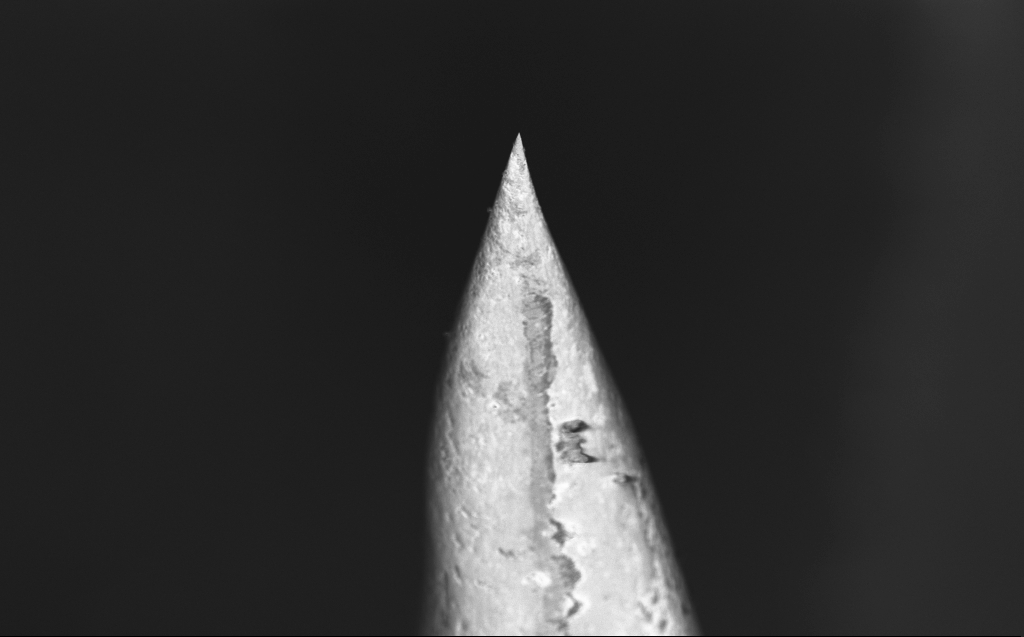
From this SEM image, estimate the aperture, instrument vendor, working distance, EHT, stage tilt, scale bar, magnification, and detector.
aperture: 30 µm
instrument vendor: Zeiss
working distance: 4 mm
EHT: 10 kV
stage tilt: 40°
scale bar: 100000 nm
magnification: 0.695 K X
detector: InLens